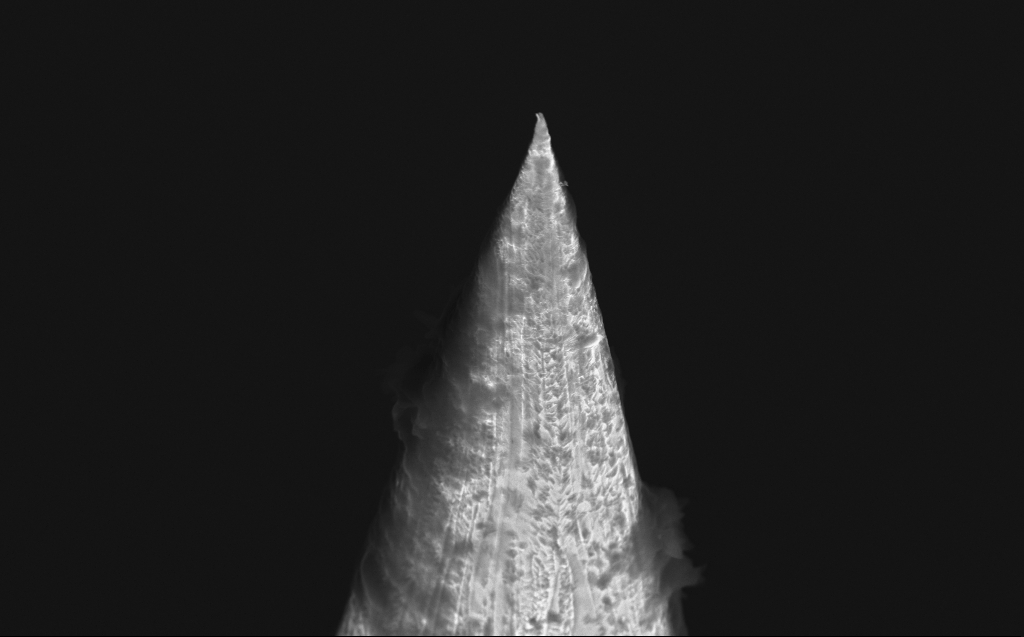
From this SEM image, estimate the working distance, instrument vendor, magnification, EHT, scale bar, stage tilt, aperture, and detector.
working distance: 4 mm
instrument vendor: Zeiss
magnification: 17.44 K X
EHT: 10 kV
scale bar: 2000 nm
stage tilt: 40°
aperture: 30 µm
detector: InLens